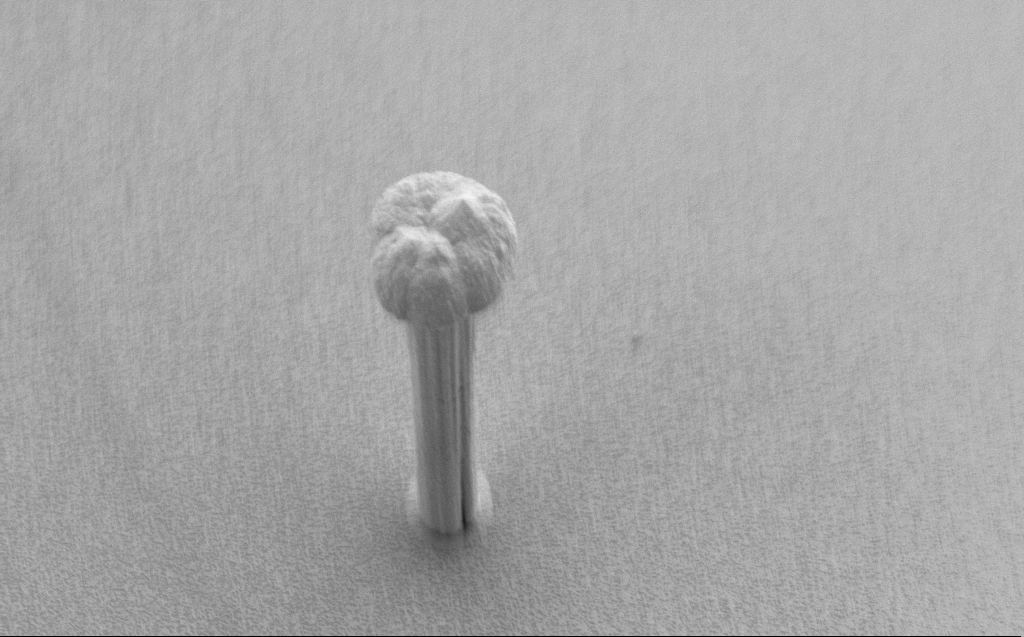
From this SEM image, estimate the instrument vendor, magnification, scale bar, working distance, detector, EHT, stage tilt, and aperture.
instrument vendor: Zeiss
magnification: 10.51 K X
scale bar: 2000 nm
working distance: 7 mm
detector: SE2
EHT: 3 kV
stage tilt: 45.1°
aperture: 120 µm